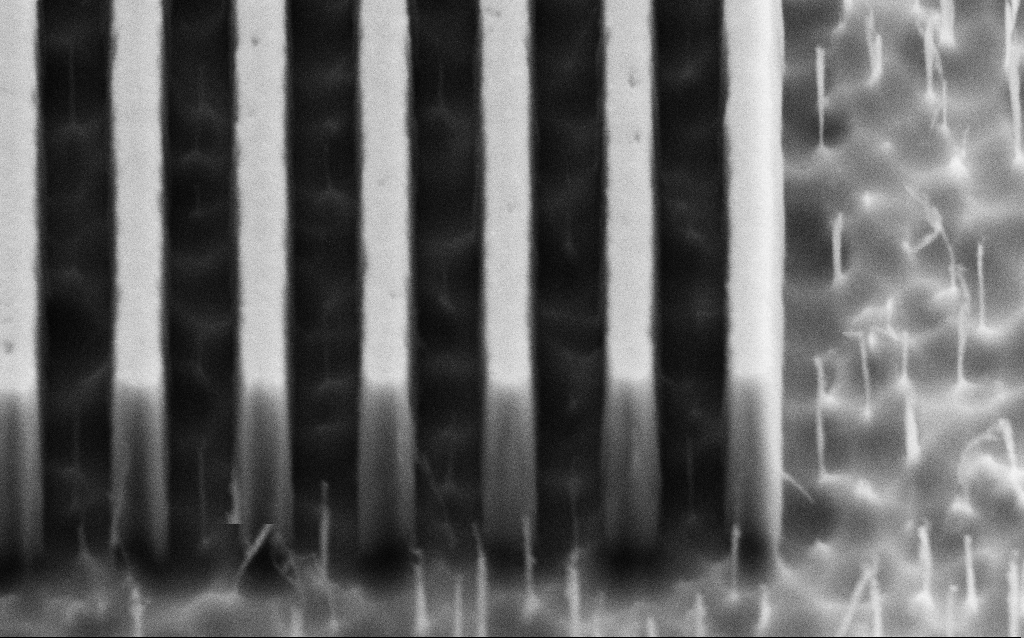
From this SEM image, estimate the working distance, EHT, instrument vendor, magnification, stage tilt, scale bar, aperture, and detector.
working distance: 5 mm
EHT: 3 kV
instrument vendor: Zeiss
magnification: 112.48 K X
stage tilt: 45°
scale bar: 200 nm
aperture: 30 µm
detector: SE2